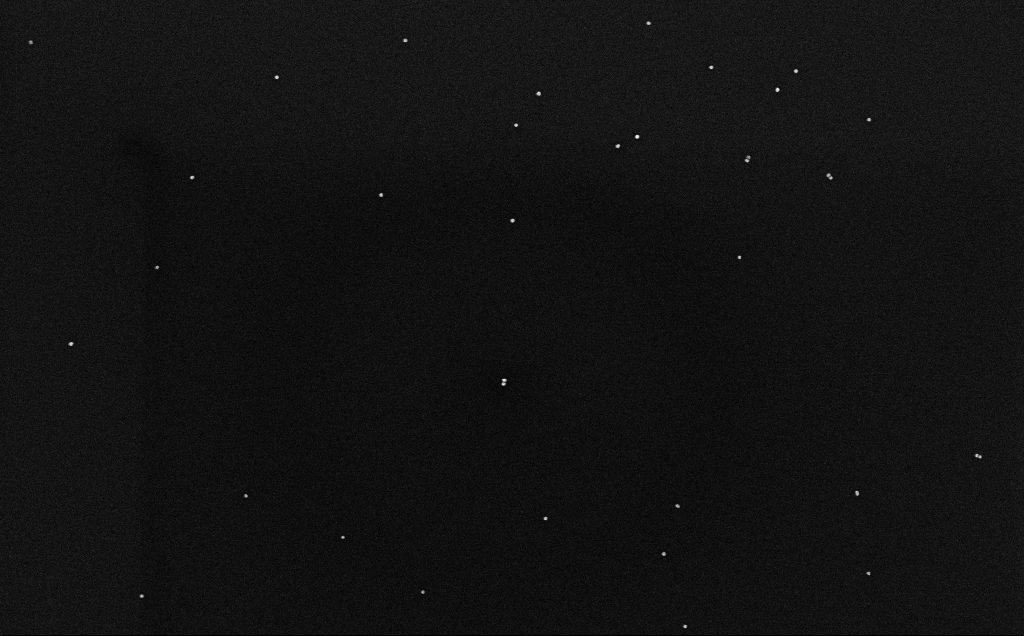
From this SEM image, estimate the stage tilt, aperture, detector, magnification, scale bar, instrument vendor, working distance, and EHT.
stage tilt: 0°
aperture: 30 µm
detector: InLens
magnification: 100 K X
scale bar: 200 nm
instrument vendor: Zeiss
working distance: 3.2 mm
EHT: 10 kV